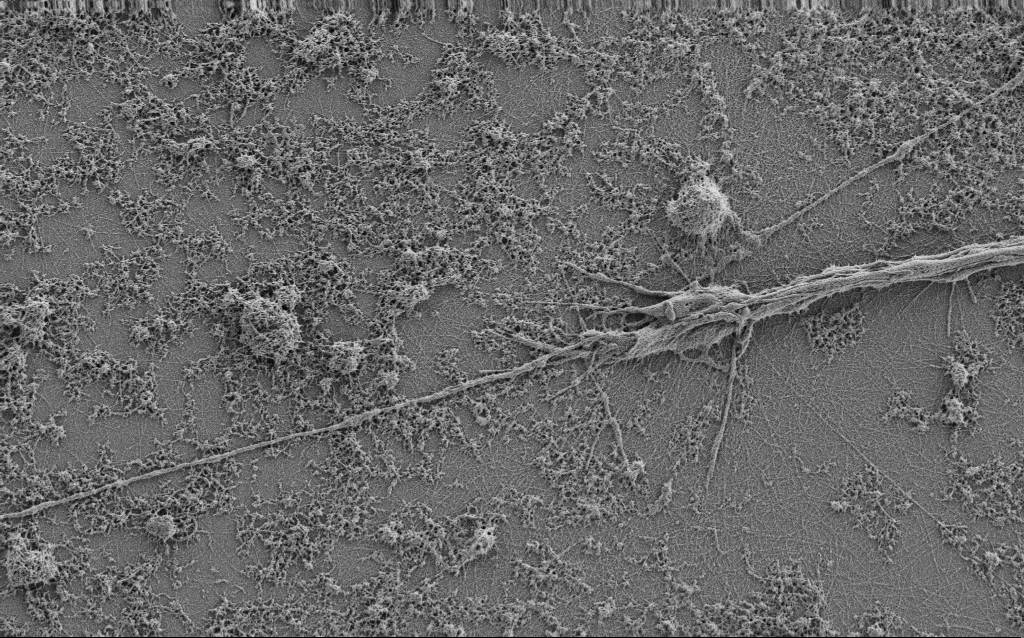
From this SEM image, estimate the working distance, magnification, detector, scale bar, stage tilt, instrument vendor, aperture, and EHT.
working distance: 4 mm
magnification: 7.5 K X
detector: SE2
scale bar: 2000 nm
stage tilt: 0°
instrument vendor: Zeiss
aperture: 30 µm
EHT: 0.9 kV